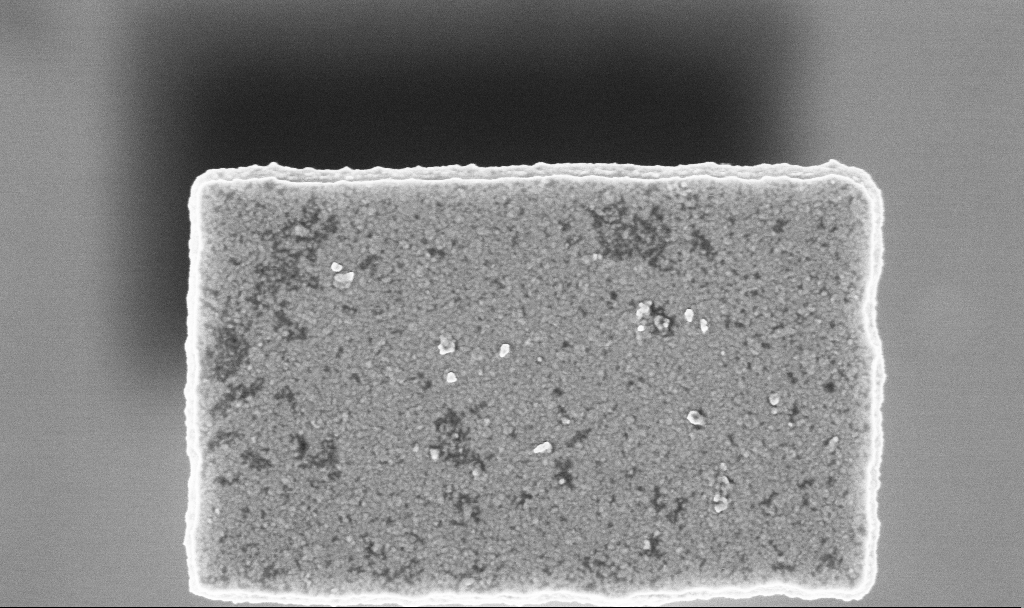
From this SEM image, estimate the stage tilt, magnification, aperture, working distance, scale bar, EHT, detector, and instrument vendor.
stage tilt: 0°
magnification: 85.46 K X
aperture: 30 µm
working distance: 3 mm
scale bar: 200 nm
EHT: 3 kV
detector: InLens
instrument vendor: Zeiss